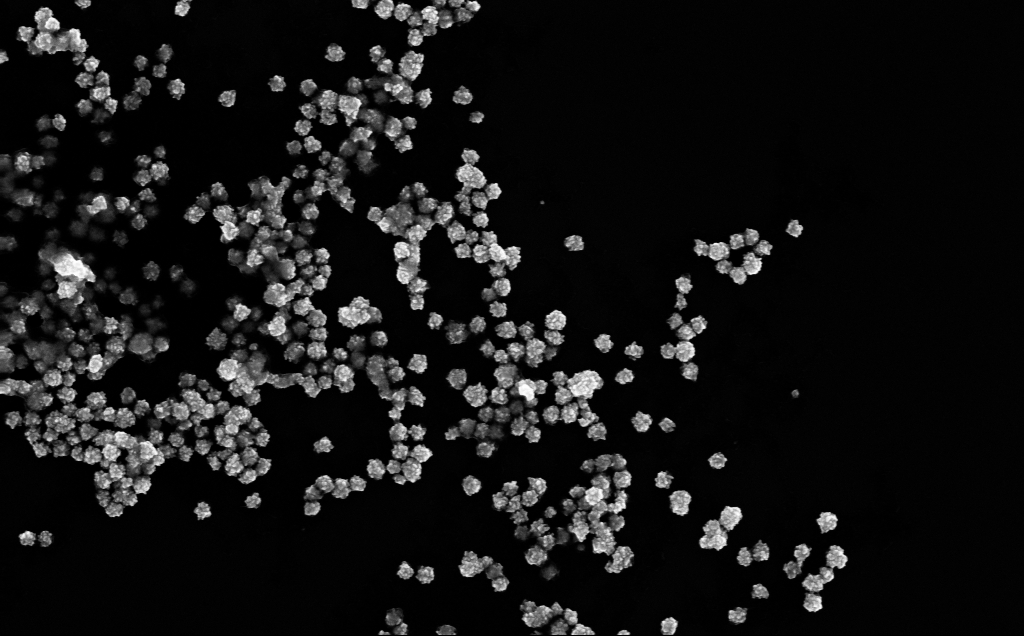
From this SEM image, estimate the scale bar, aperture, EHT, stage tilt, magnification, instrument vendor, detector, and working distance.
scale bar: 200 nm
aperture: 30 µm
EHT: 10 kV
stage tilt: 0°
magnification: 99.71 K X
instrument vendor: Zeiss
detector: InLens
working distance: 3 mm